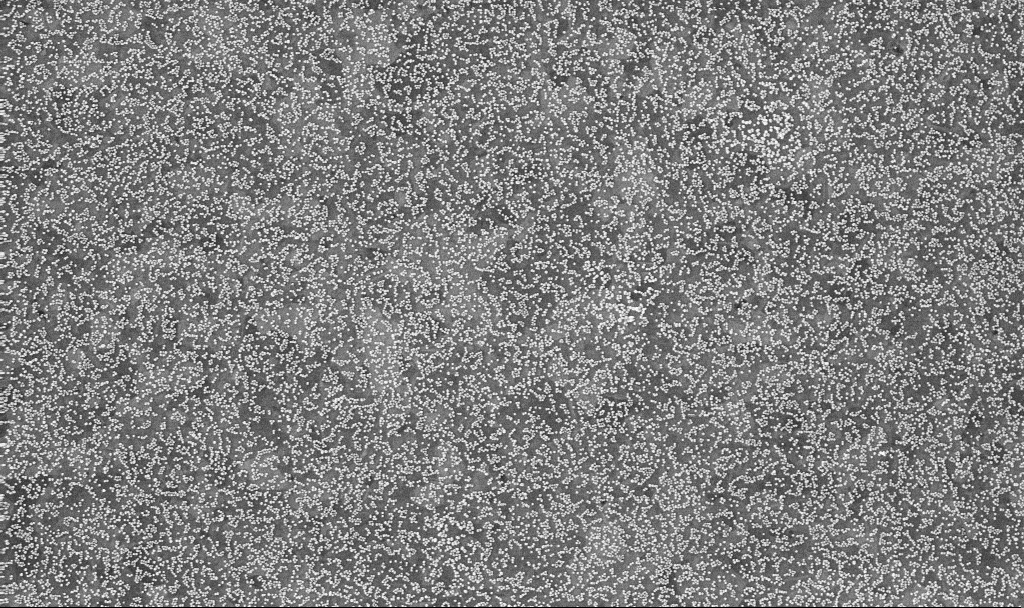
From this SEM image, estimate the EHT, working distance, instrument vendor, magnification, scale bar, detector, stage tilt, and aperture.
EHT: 10 kV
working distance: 3.3 mm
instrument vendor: Zeiss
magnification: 50 K X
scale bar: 1000 nm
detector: InLens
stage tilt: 0°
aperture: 30 µm